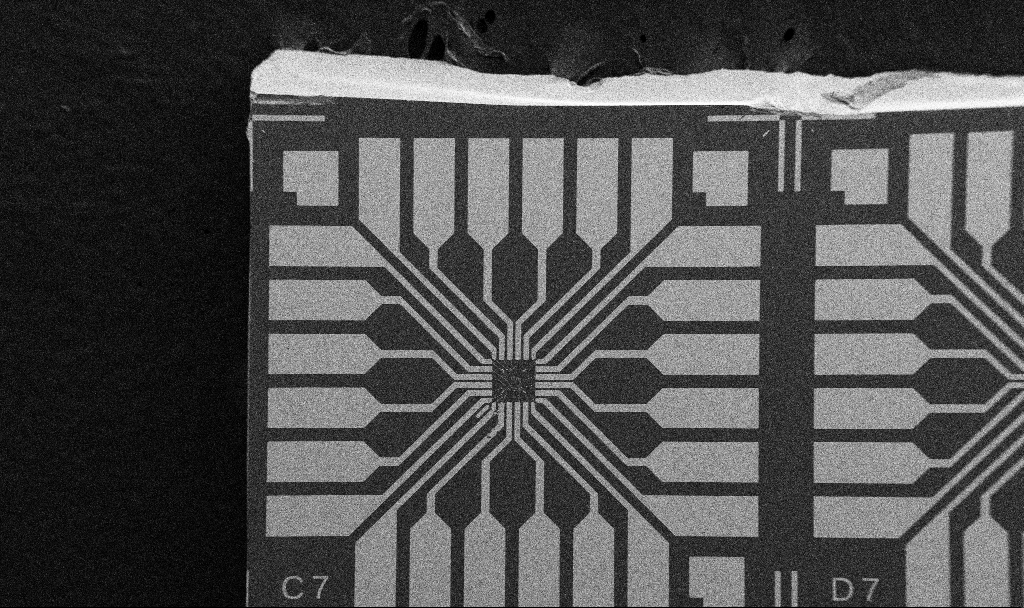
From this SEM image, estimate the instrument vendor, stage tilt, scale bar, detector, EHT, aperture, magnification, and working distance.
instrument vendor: Zeiss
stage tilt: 0°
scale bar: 200000 nm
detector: SE2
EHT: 5 kV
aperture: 30 µm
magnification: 0.1 K X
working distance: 10.7 mm